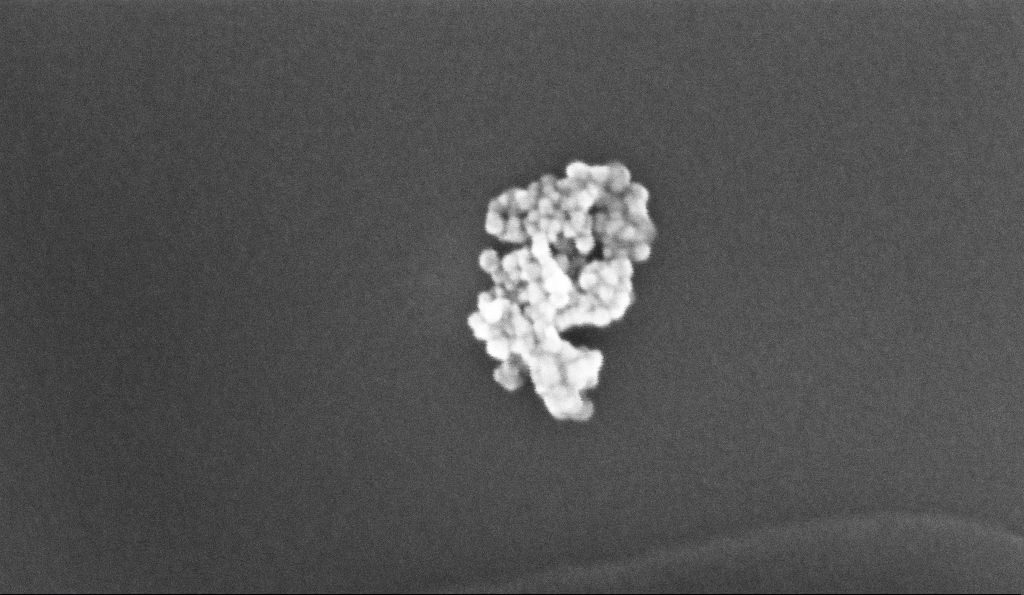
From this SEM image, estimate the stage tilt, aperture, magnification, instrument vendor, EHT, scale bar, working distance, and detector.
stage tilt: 0°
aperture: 30 µm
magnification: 381.35 K X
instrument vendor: Zeiss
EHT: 5 kV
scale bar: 100 nm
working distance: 3.1 mm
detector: InLens